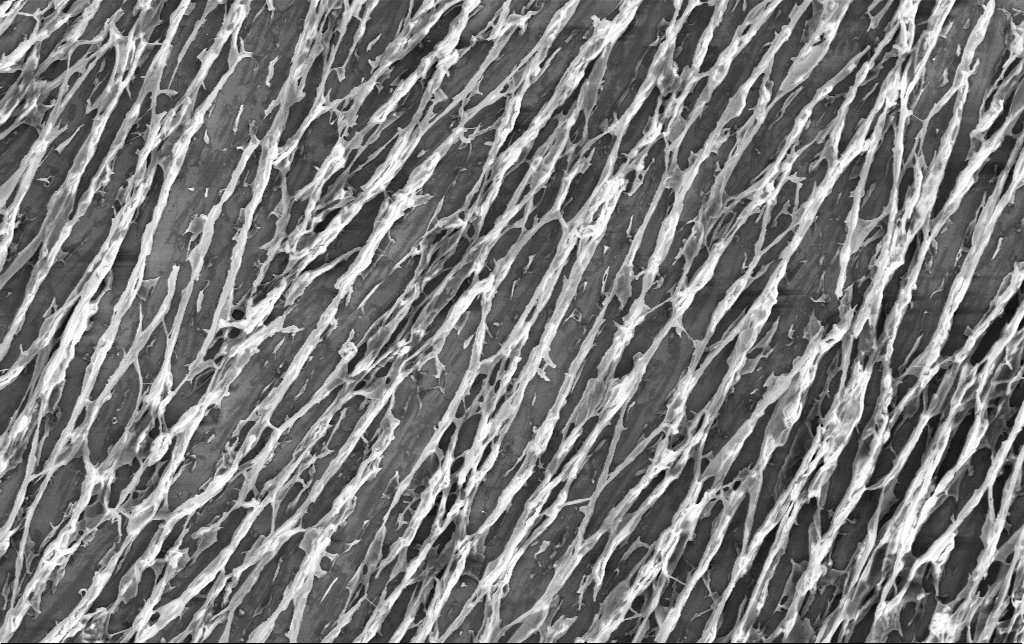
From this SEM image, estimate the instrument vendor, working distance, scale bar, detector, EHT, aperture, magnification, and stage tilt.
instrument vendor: Zeiss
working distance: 3.2 mm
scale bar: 10000 nm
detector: InLens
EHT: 3 kV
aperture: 30 µm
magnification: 6.54 K X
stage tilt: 0°